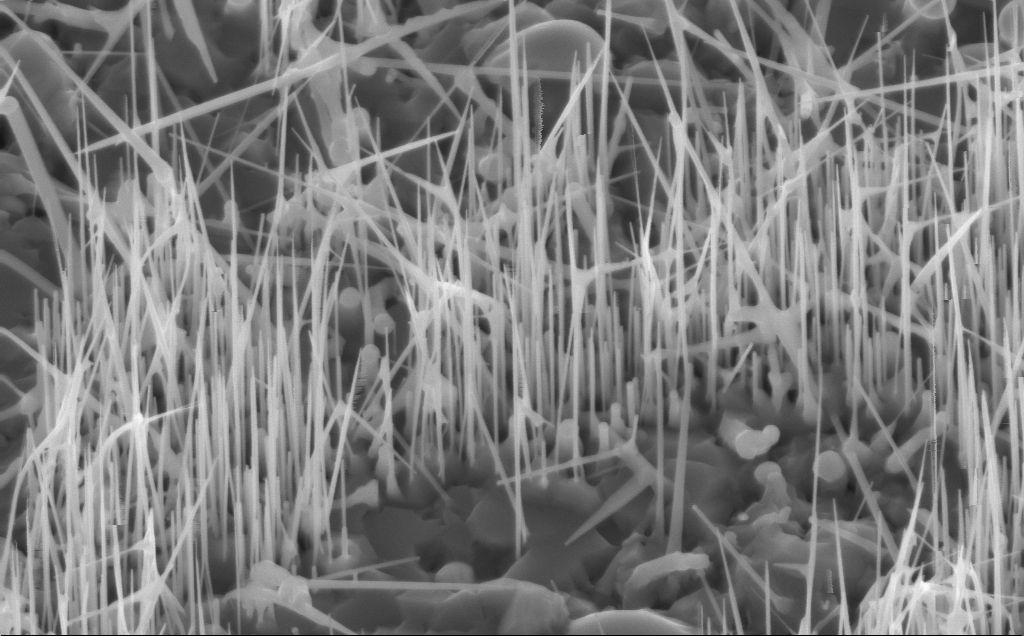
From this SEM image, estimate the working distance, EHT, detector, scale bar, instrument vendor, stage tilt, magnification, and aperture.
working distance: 5 mm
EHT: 10 kV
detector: InLens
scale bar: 1000 nm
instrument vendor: Zeiss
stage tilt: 30°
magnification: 40 K X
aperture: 30 µm